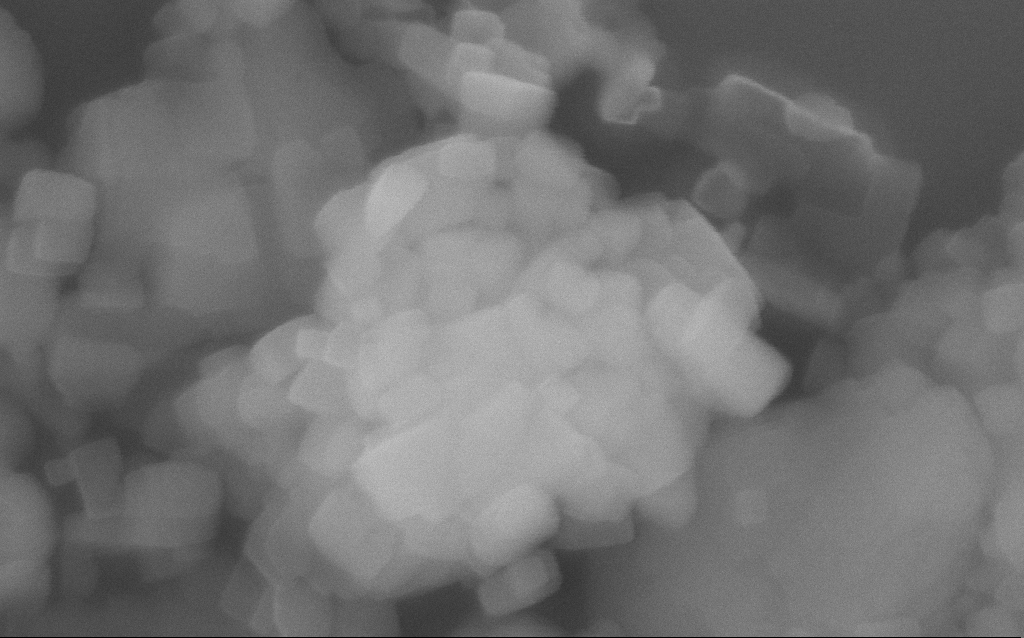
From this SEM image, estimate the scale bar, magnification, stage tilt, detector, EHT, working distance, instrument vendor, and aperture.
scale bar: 200 nm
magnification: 236.95 K X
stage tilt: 0°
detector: InLens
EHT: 10 kV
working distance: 3 mm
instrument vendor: Zeiss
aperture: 30 µm